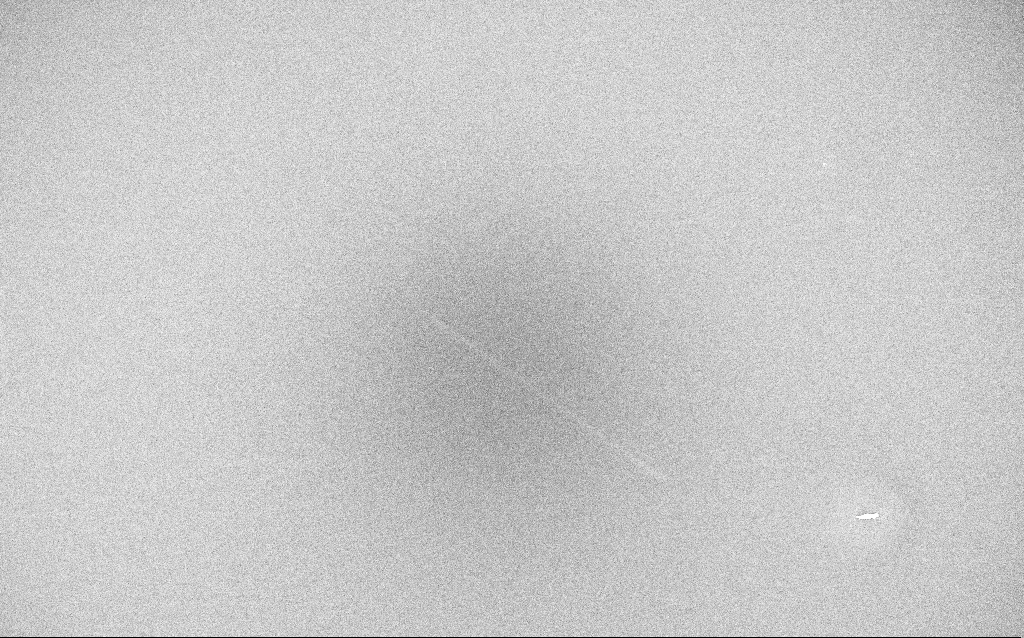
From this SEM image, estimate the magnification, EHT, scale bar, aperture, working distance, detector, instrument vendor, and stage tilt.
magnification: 0.25 K X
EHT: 20 kV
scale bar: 100000 nm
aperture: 30 µm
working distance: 1.9 mm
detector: InLens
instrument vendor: Zeiss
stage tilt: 0°